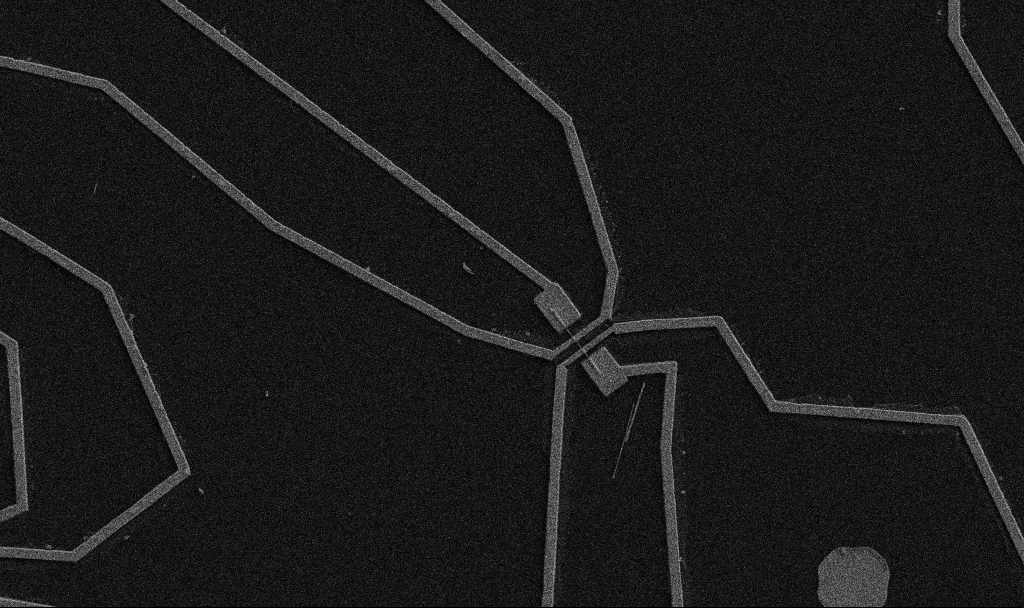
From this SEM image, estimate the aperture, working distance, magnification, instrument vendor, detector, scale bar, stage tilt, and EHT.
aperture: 30 µm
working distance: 10.7 mm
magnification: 5 K X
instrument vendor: Zeiss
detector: SE2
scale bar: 10000 nm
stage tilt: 0°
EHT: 5 kV